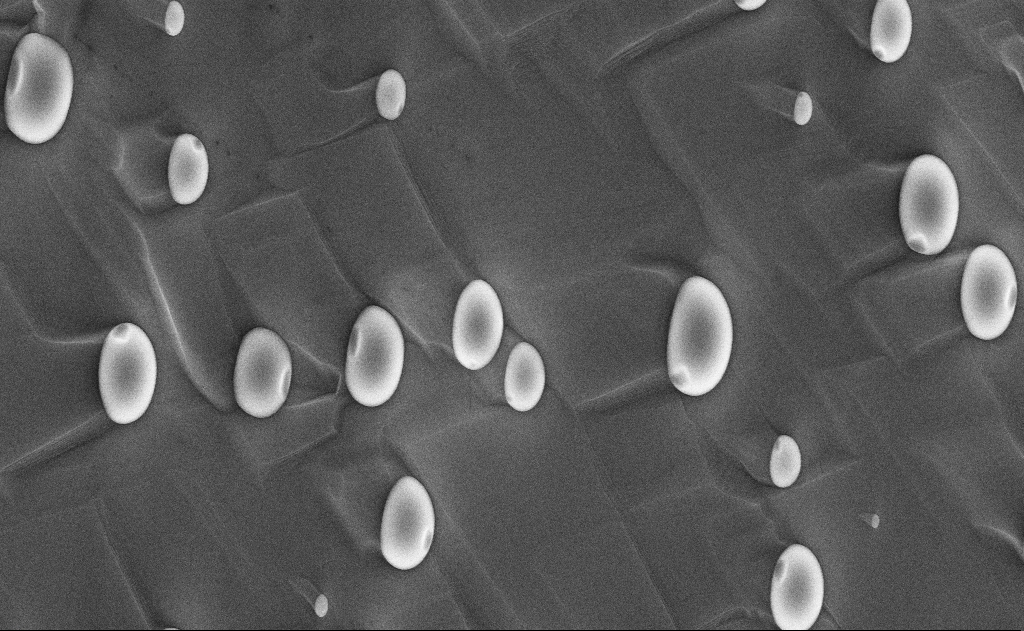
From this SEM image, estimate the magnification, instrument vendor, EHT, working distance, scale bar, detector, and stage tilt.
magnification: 20 K X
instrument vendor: Zeiss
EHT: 10 kV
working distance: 15 mm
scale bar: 1000 nm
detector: InLens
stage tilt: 0°